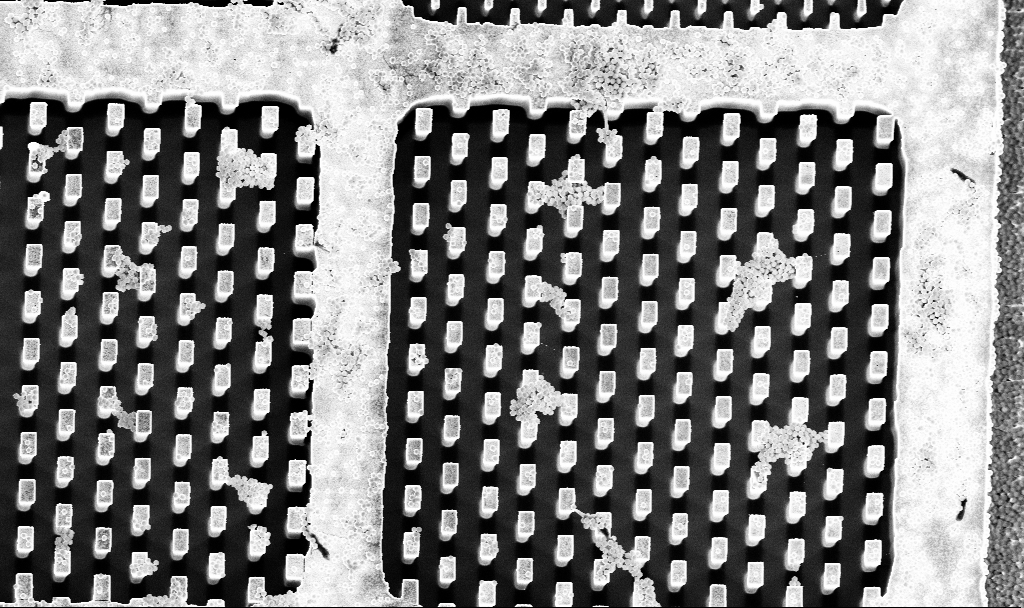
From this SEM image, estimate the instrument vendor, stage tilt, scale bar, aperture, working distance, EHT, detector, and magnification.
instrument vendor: Zeiss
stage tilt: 5°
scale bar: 10000 nm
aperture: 30 µm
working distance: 3.3 mm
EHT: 5 kV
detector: InLens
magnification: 3.59 K X